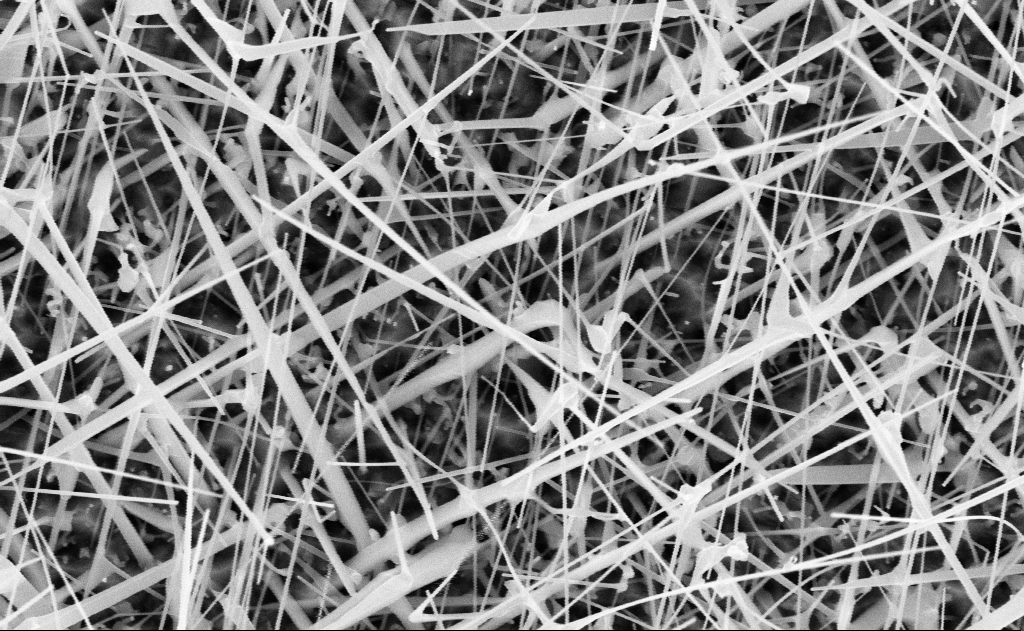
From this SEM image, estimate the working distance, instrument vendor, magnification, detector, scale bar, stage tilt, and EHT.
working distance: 20 mm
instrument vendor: Zeiss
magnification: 40 K X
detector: InLens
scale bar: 1000 nm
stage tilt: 0°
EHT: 10 kV